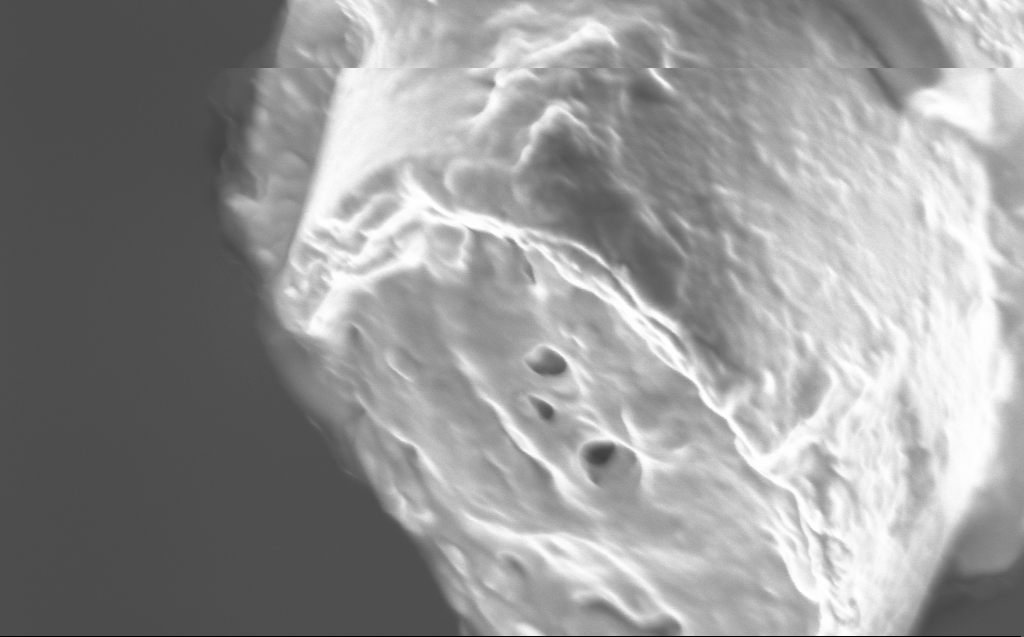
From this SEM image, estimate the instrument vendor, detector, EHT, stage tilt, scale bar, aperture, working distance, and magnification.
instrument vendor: Zeiss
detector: InLens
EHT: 1 kV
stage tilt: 45°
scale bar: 200 nm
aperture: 30 µm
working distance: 2 mm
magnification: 102.8 K X